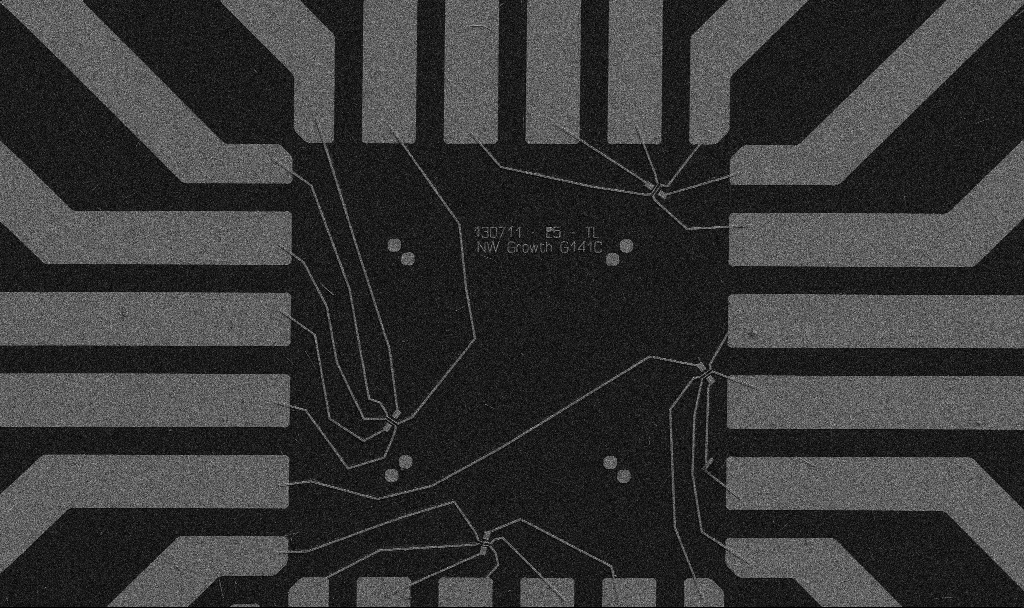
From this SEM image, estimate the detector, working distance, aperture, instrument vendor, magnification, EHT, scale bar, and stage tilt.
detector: SE2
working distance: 10.7 mm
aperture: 30 µm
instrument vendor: Zeiss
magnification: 1 K X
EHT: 5 kV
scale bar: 20000 nm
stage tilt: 0°